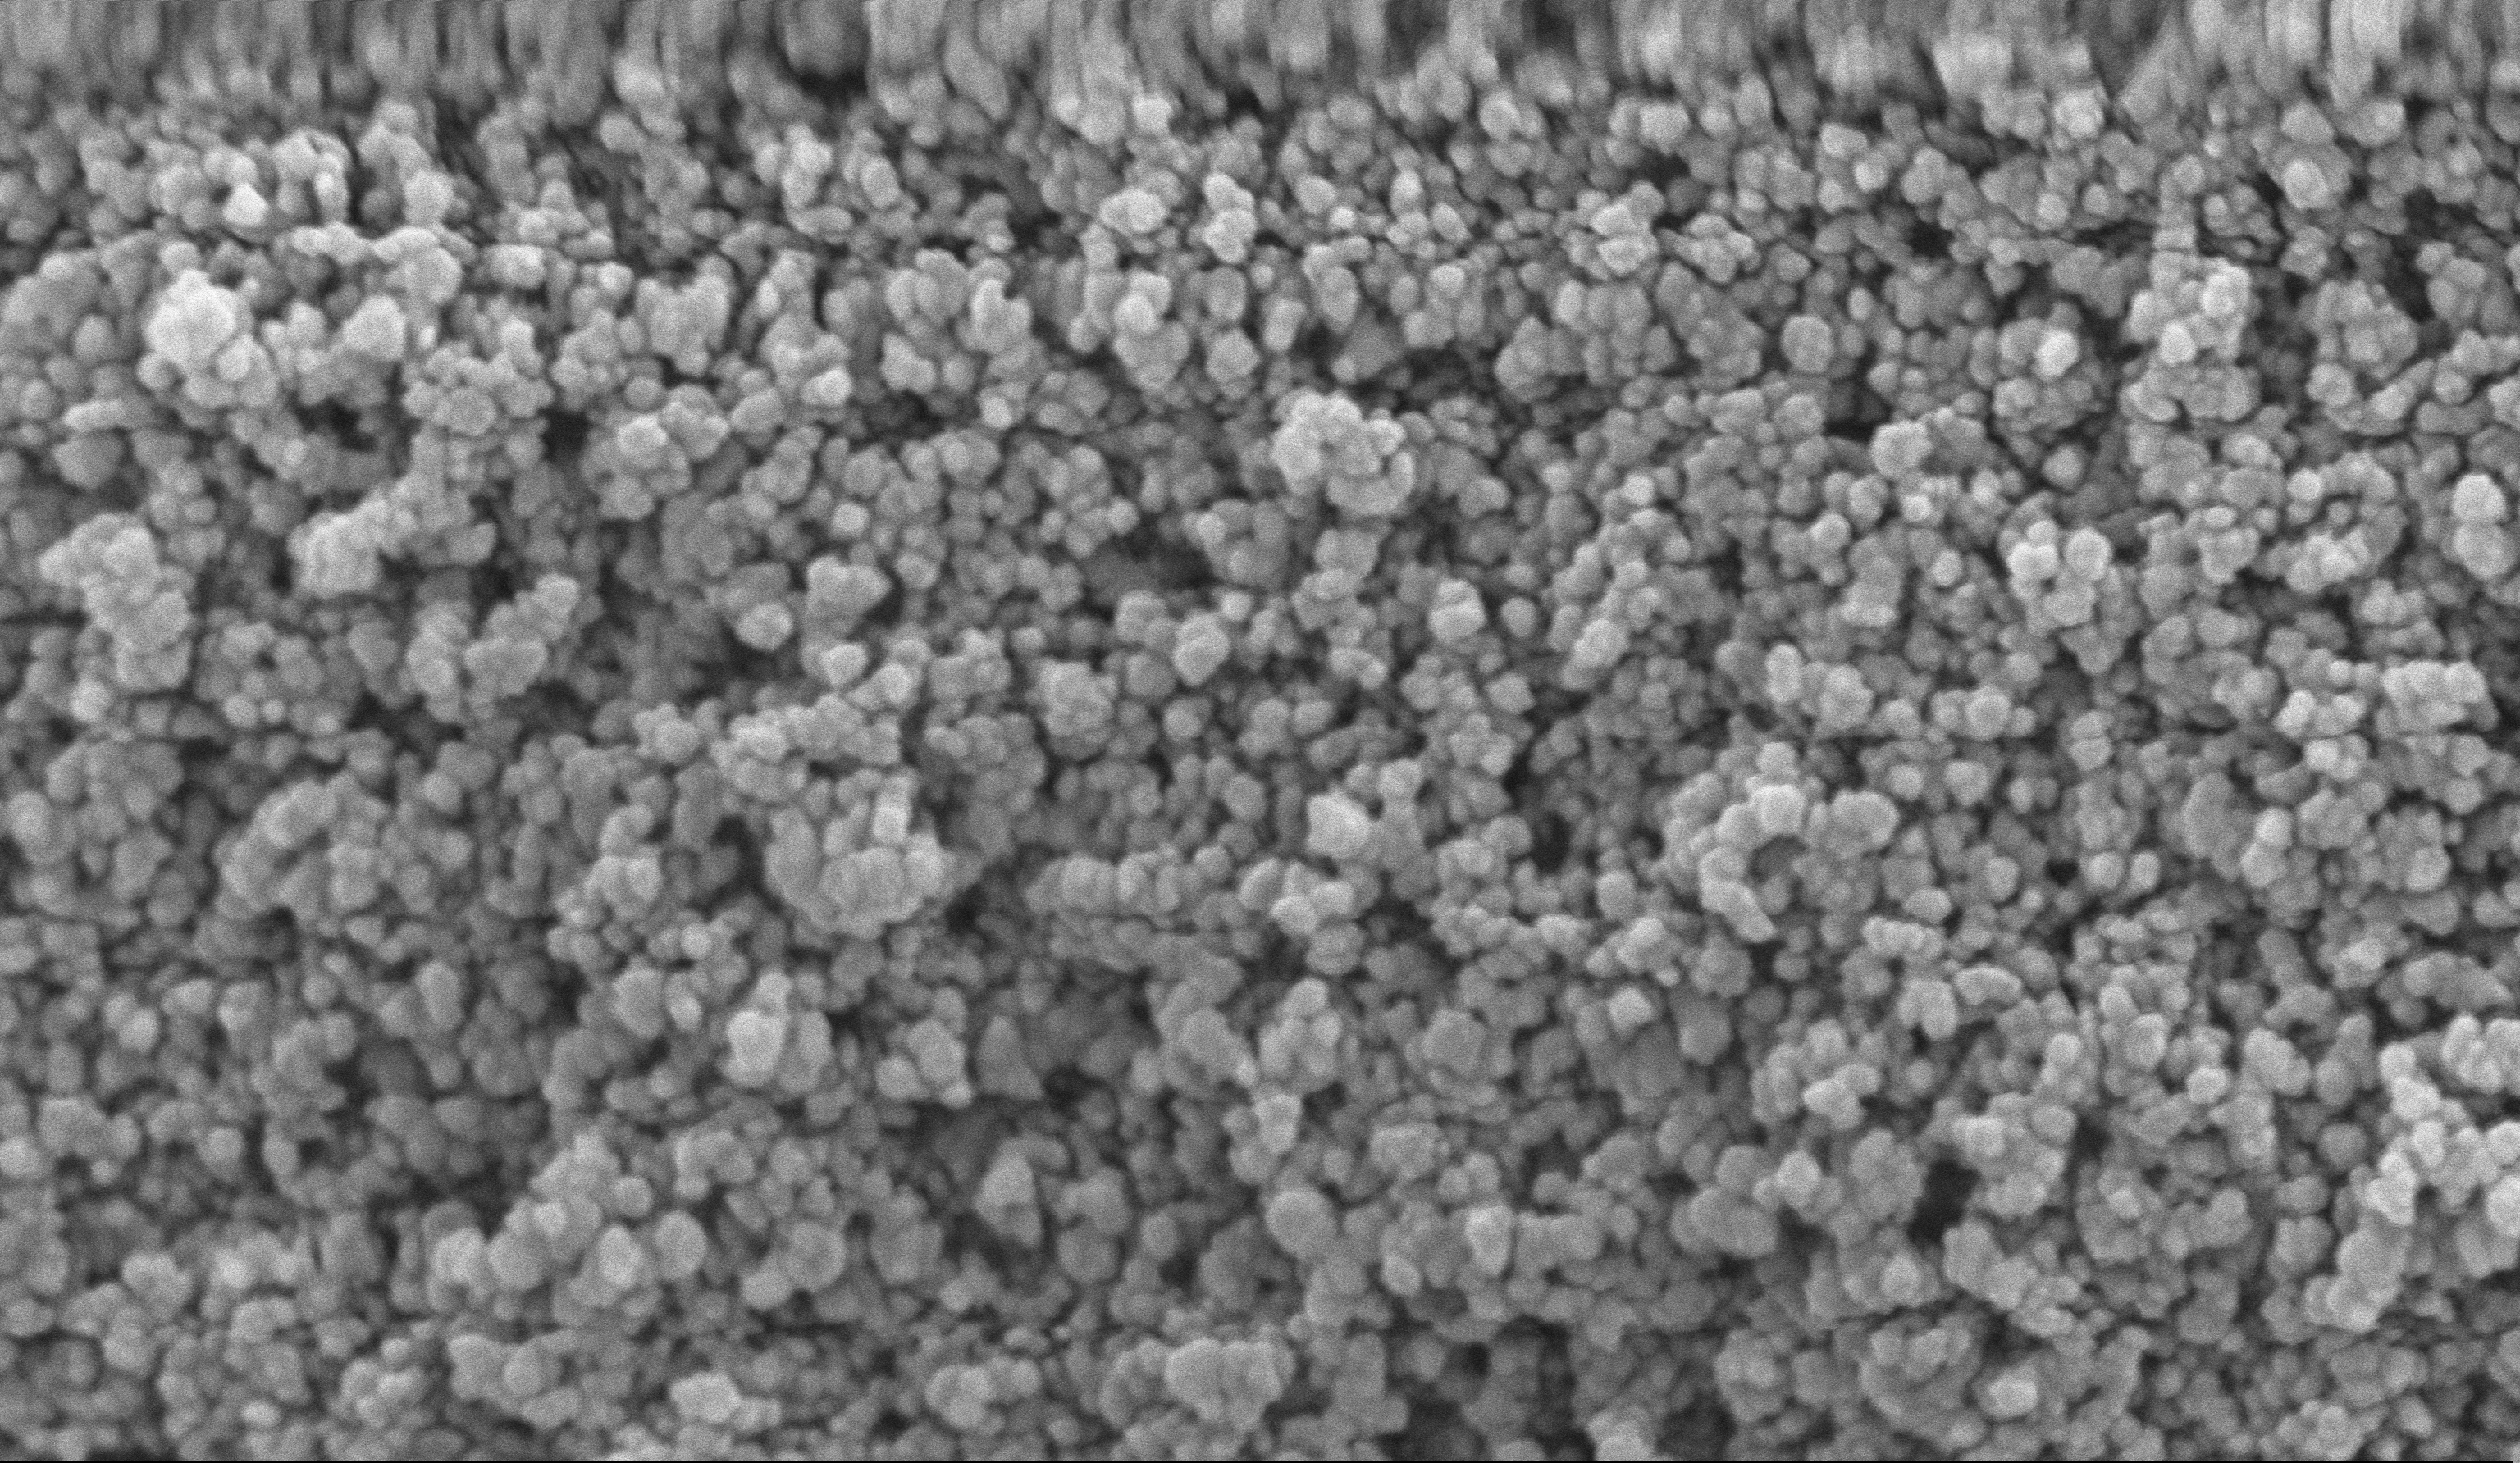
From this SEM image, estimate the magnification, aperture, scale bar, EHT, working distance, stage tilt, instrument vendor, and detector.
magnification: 135 K X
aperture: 30 µm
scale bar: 100 nm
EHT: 10 kV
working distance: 5.1 mm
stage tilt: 0°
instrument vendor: Zeiss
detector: InLens